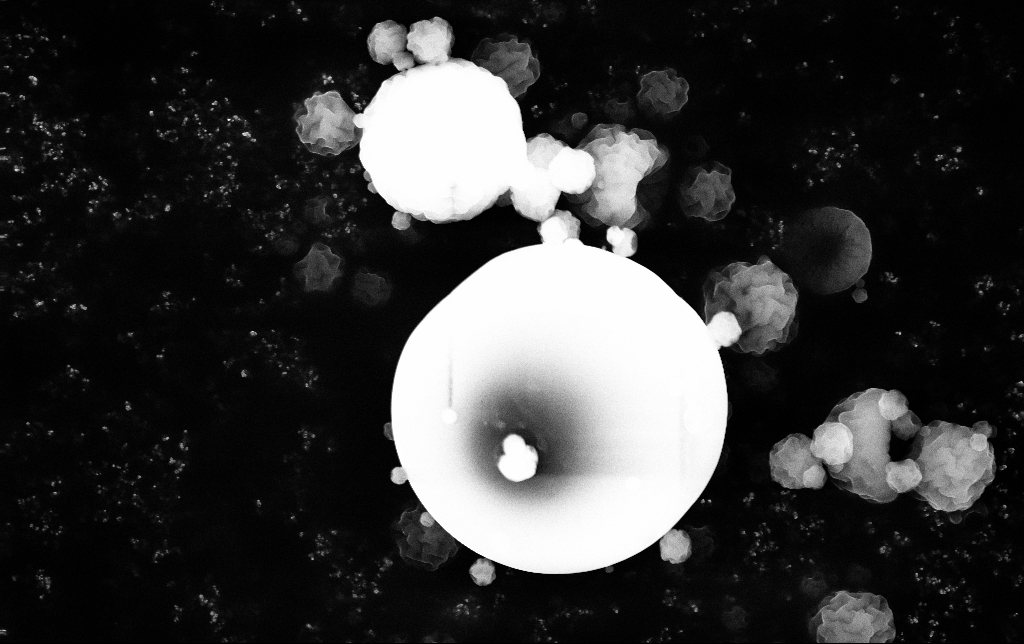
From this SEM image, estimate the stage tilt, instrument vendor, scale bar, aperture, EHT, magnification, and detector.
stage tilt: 0°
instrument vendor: Zeiss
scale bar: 2000 nm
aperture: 30 µm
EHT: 15 kV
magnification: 15.18 K X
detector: InLens